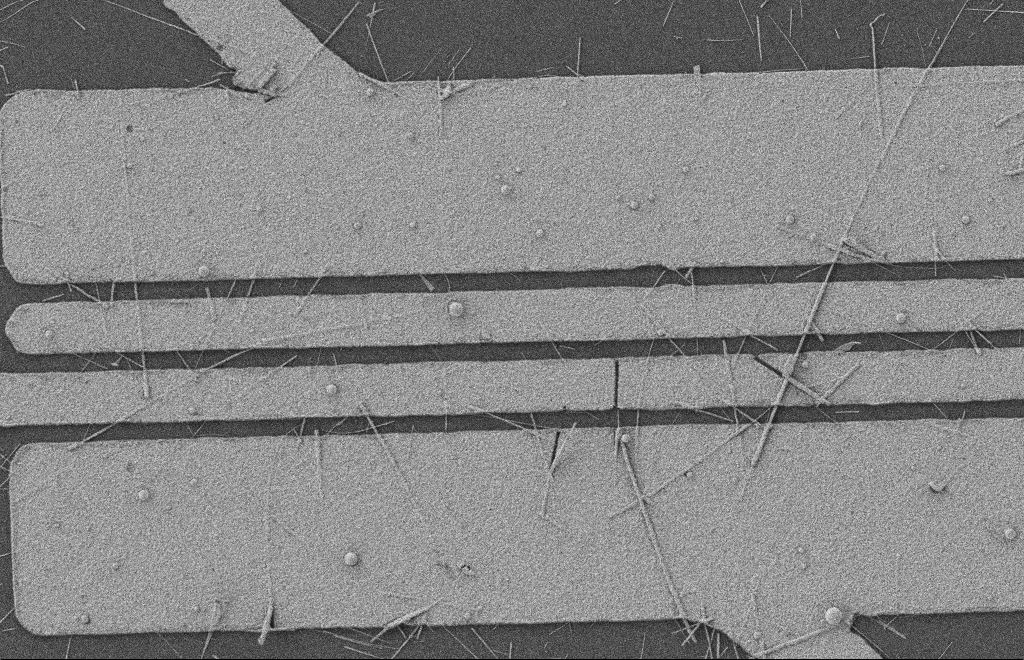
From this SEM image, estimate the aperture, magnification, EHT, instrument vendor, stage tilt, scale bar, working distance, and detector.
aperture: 20 µm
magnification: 6.41 K X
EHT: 2 kV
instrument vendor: Zeiss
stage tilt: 0°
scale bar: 2000 nm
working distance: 8 mm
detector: SE2